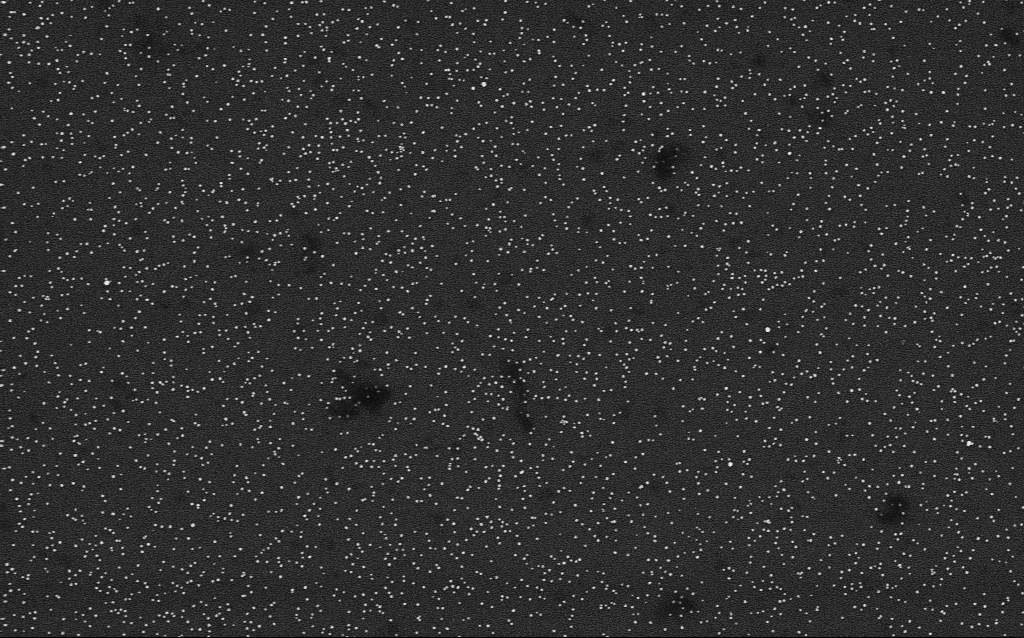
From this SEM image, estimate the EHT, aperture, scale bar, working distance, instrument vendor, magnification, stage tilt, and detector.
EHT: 4 kV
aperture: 30 µm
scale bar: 1000 nm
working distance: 2.1 mm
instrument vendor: Zeiss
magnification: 50 K X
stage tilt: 0°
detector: InLens